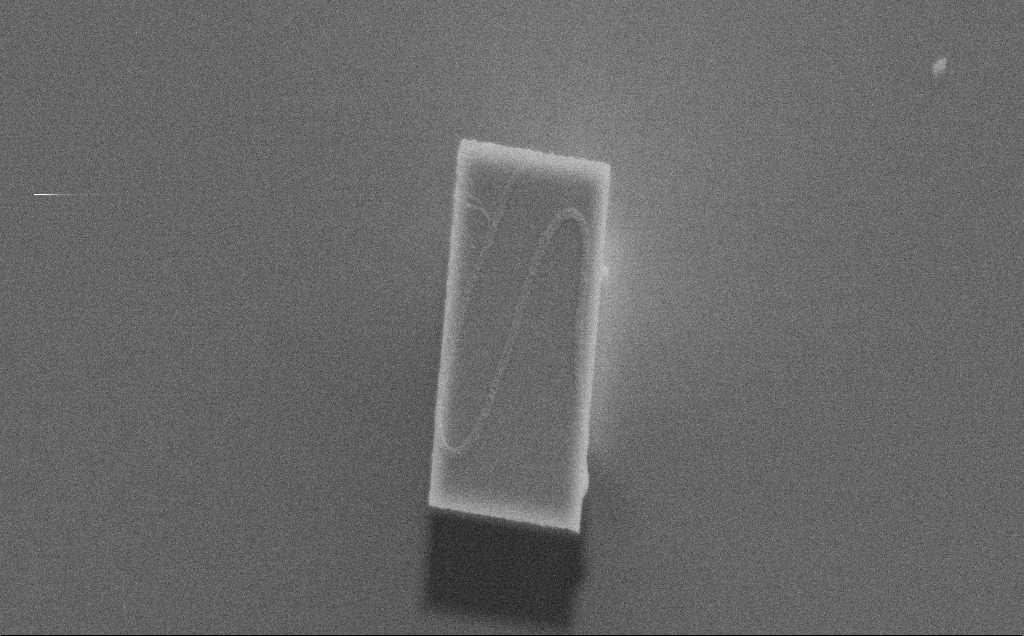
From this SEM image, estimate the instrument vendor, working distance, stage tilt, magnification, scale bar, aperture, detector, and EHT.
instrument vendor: Zeiss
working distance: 4 mm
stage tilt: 0°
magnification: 19.62 K X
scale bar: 1000 nm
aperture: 30 µm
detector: SE2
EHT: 5 kV